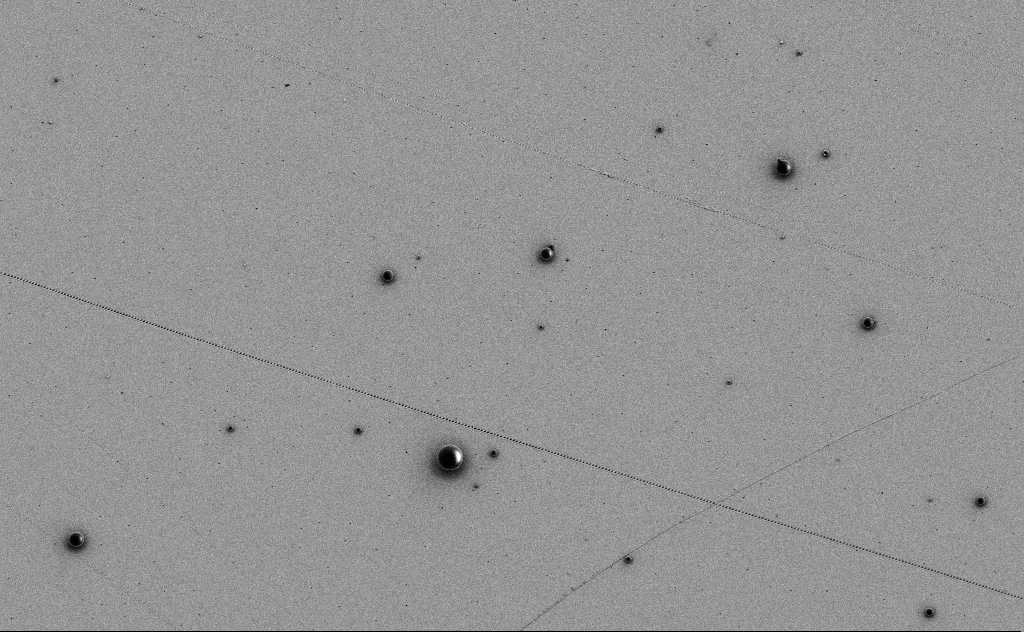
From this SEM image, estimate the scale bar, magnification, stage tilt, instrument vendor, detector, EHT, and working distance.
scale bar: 10000 nm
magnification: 3.33 K X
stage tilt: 0°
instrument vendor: Zeiss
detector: SE2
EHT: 3 kV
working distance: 10 mm